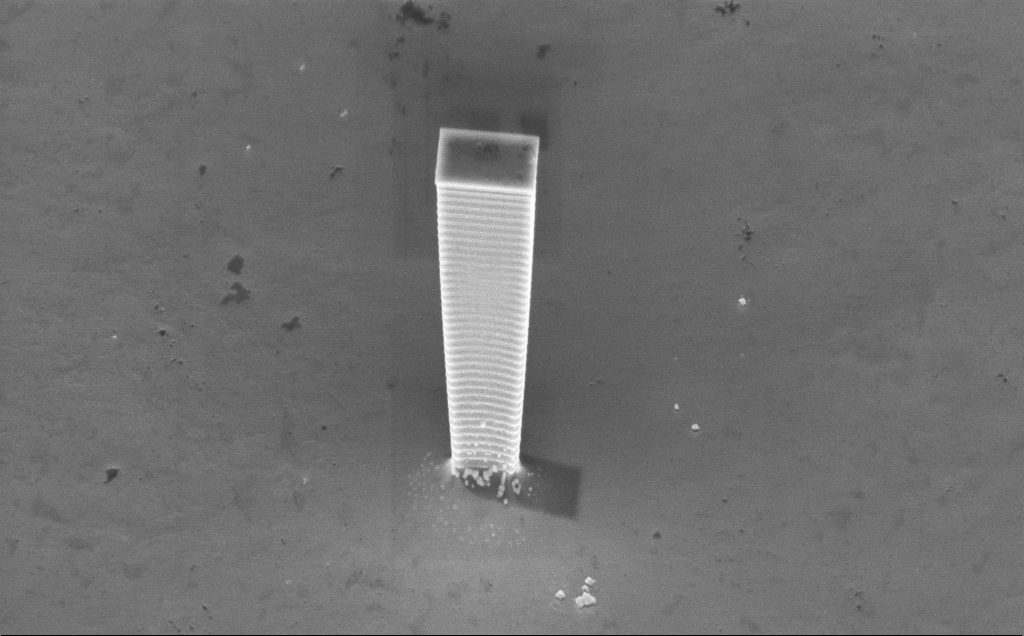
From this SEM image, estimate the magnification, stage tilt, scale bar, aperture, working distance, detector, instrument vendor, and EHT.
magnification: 7.52 K X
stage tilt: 45°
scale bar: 2000 nm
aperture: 30 µm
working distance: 5 mm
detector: InLens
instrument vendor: Zeiss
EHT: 7.5 kV